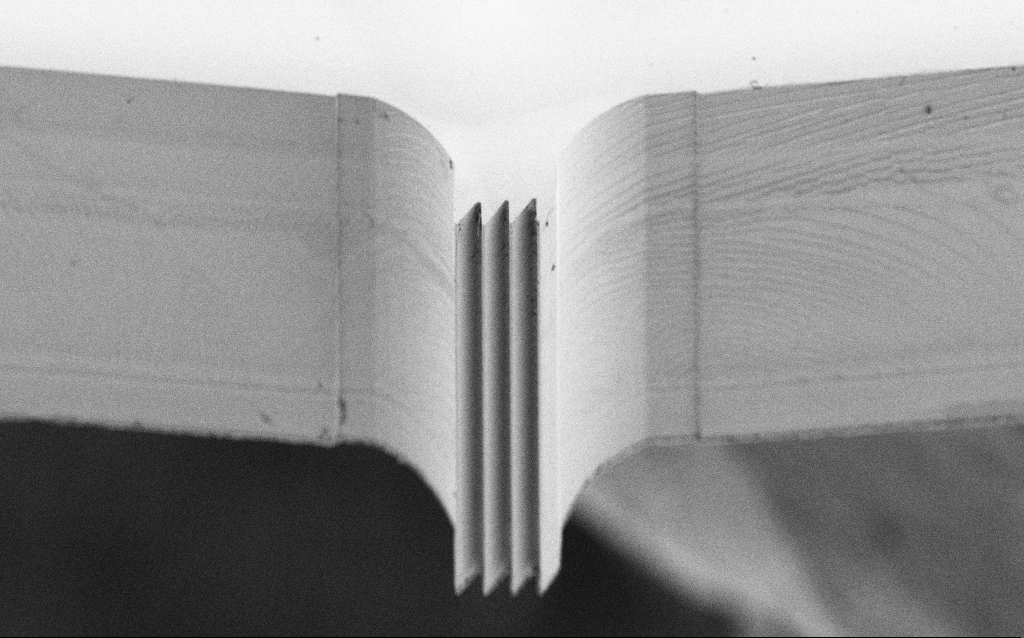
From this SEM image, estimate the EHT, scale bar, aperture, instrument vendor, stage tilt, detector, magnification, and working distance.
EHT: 5 kV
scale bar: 20000 nm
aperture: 30 µm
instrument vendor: Zeiss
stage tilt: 45°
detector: SE2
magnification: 0.904 K X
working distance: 6 mm